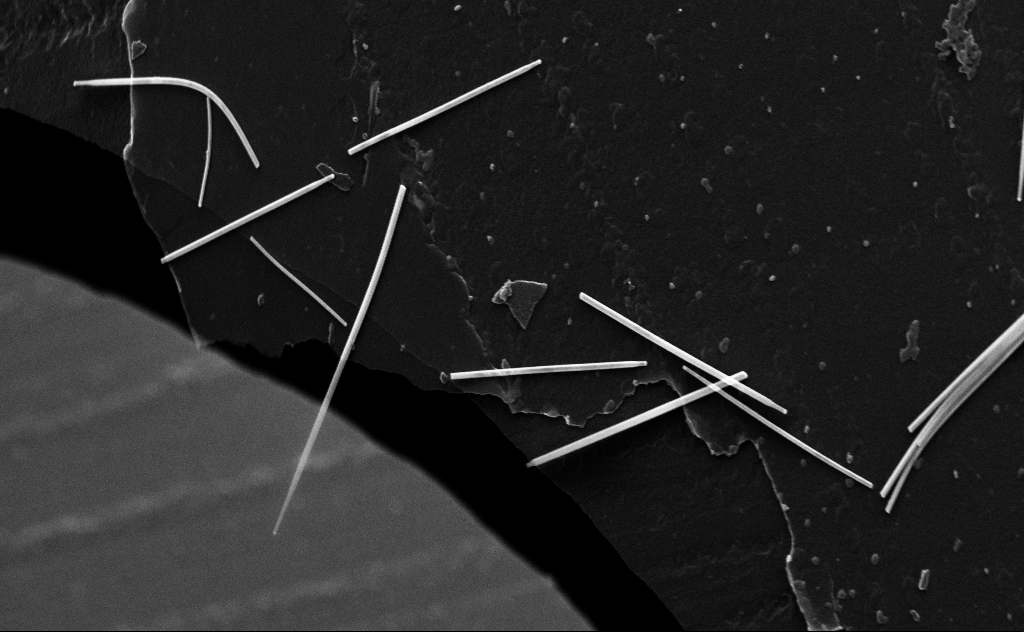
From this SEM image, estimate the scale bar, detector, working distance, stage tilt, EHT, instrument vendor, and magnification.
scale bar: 1000 nm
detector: InLens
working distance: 8 mm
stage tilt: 0°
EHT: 20 kV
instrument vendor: Zeiss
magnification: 52.58 K X